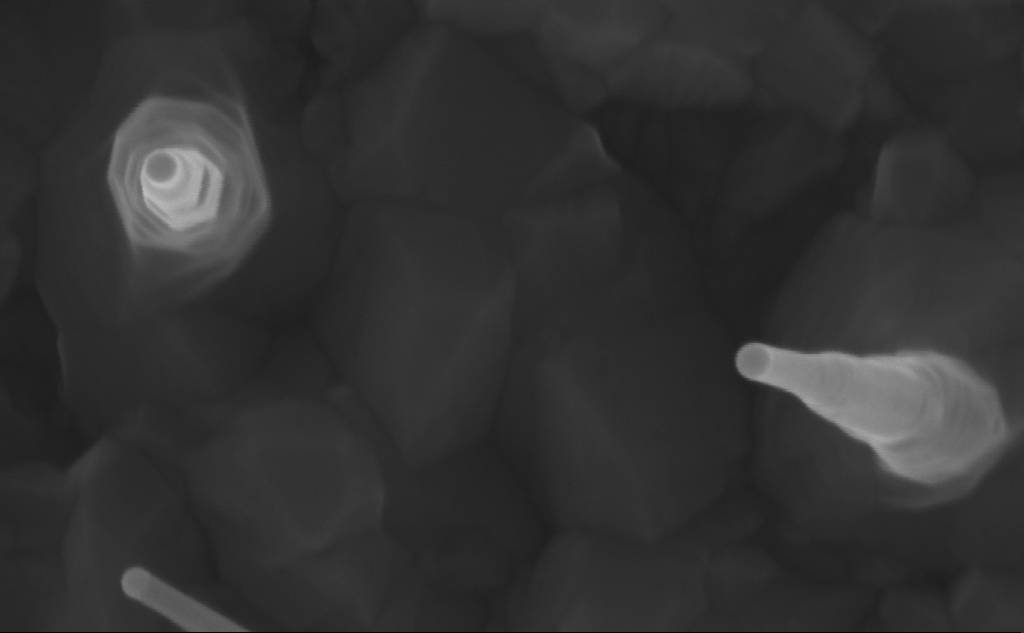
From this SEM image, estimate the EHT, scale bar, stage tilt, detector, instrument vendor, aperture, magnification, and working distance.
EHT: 10 kV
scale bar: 100 nm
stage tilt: -0°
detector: InLens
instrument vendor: Zeiss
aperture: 30 µm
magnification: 282.29 K X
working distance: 7 mm